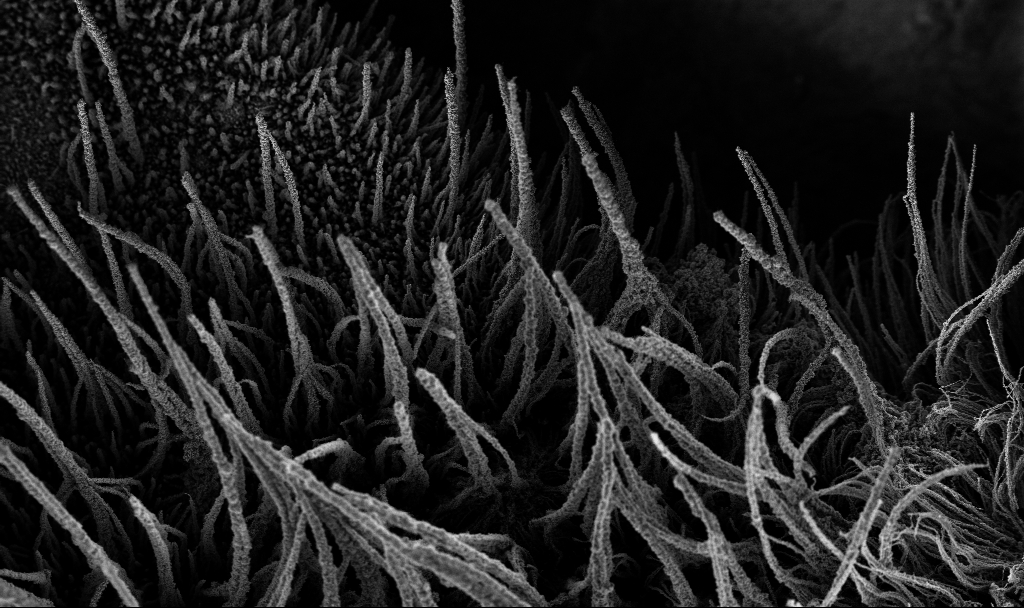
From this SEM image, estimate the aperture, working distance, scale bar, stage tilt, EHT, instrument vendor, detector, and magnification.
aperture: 30 µm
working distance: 3.8 mm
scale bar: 100000 nm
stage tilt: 0°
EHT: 3 kV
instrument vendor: Zeiss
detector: InLens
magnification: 0.25 K X